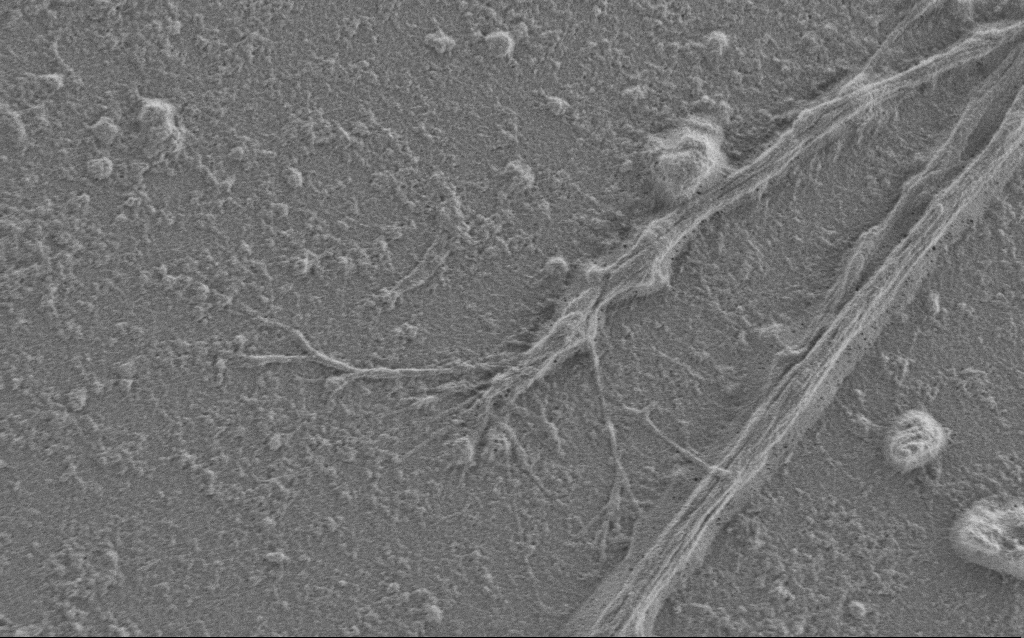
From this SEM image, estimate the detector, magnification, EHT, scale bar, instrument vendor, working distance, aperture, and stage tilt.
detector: SE2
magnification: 7.5 K X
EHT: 1 kV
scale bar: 2000 nm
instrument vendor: Zeiss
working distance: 6 mm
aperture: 30 µm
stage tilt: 0°